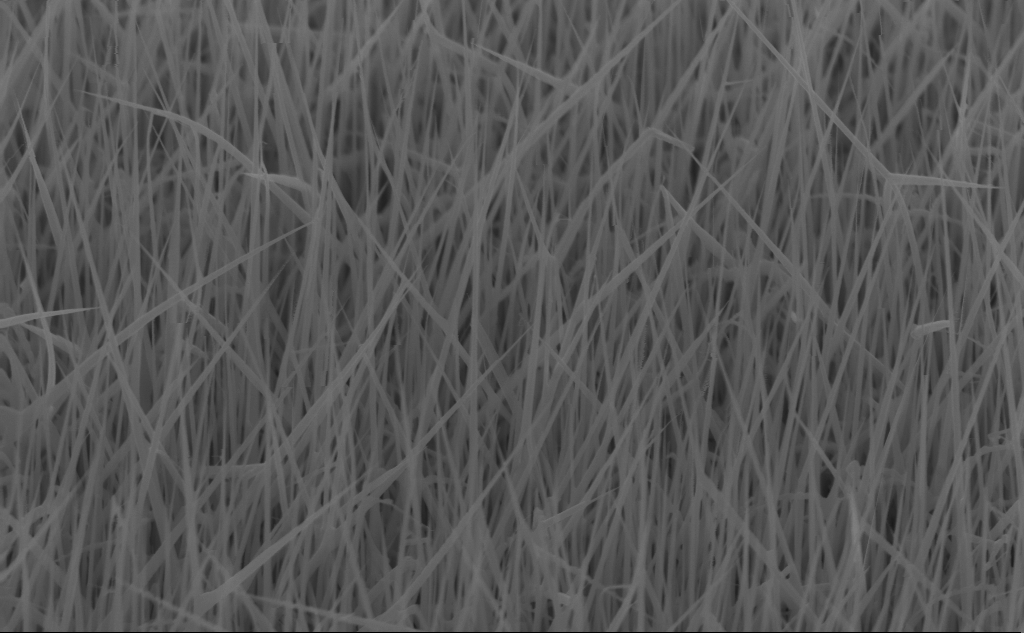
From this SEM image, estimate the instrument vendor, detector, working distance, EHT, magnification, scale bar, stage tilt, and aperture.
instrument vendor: Zeiss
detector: InLens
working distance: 4 mm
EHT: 10 kV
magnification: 40 K X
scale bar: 1000 nm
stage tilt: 45°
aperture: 30 µm